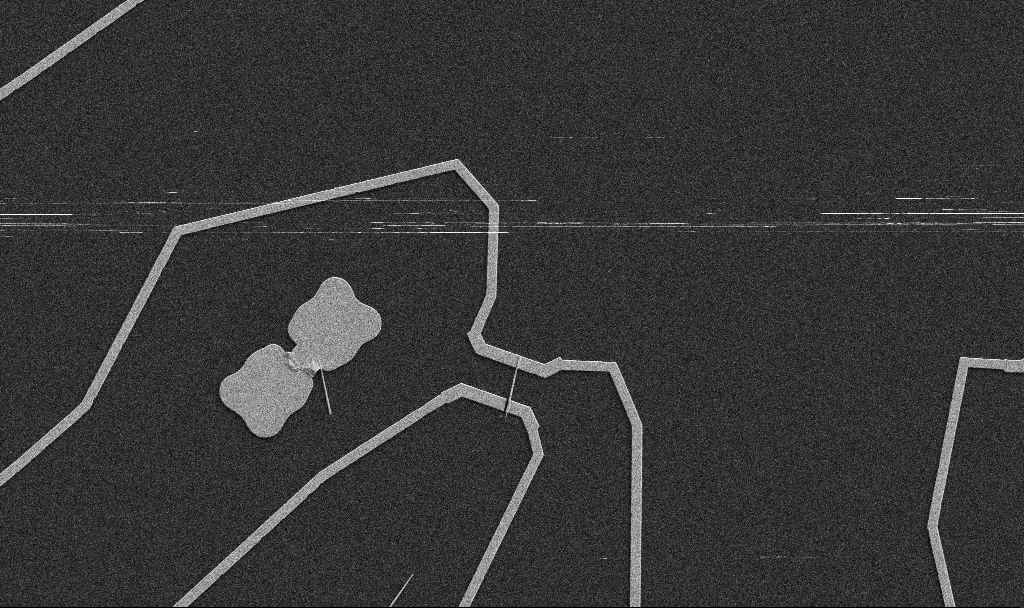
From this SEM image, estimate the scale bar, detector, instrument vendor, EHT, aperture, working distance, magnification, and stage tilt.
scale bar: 10000 nm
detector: SE2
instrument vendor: Zeiss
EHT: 5 kV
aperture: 30 µm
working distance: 10.7 mm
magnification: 5 K X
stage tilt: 0°